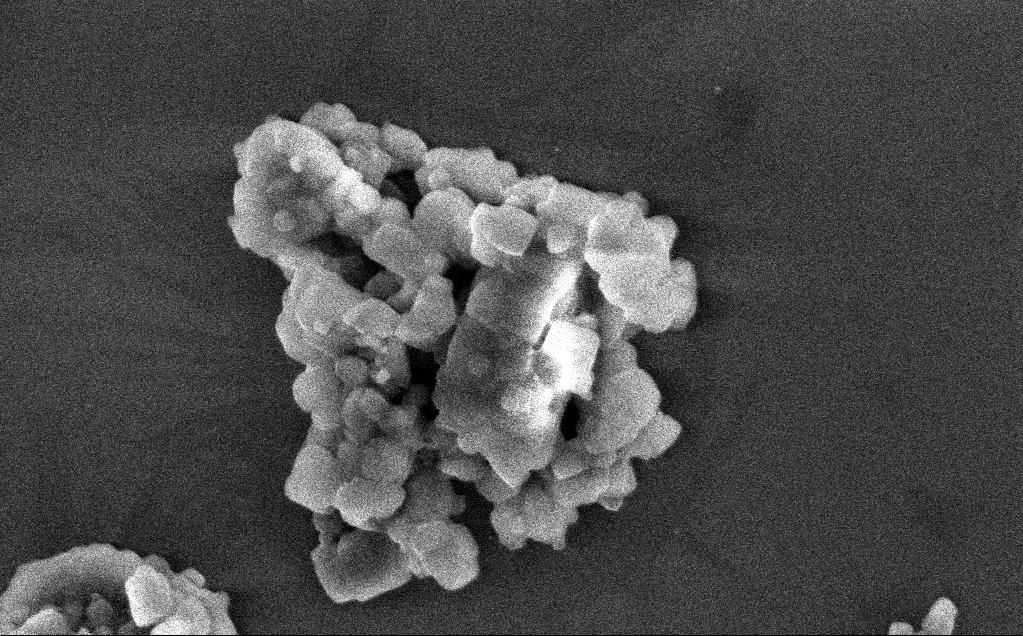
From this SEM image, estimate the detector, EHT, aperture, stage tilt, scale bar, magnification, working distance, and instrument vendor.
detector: InLens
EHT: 3 kV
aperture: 30 µm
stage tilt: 0°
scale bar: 200 nm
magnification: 124.47 K X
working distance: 3 mm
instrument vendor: Zeiss